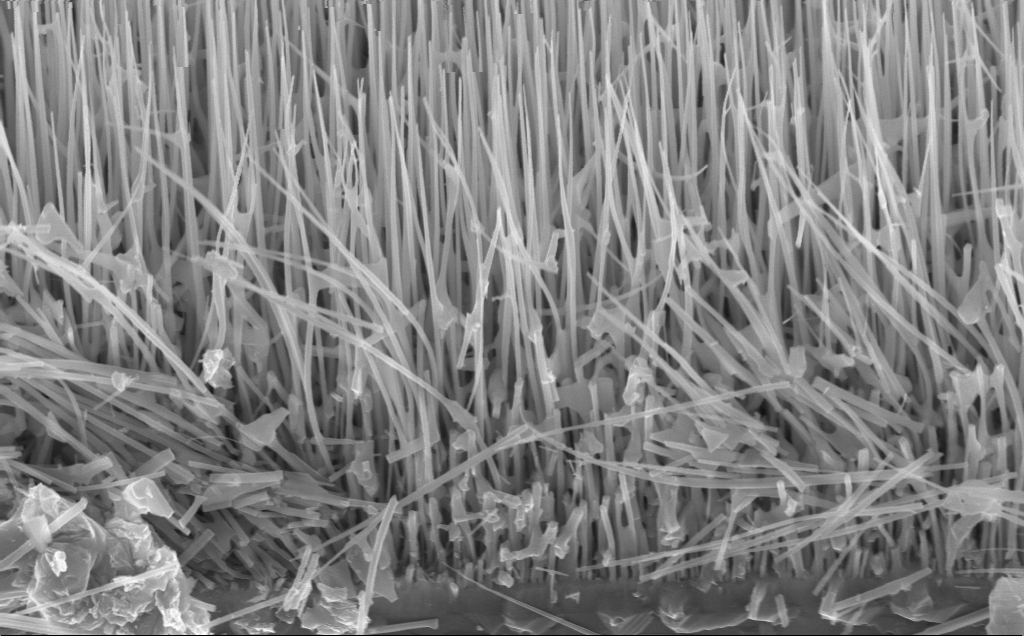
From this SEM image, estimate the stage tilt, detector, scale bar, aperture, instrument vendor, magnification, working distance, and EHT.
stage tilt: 45°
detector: InLens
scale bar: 1000 nm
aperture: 30 µm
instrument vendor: Zeiss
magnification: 39.76 K X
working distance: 7 mm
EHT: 10 kV